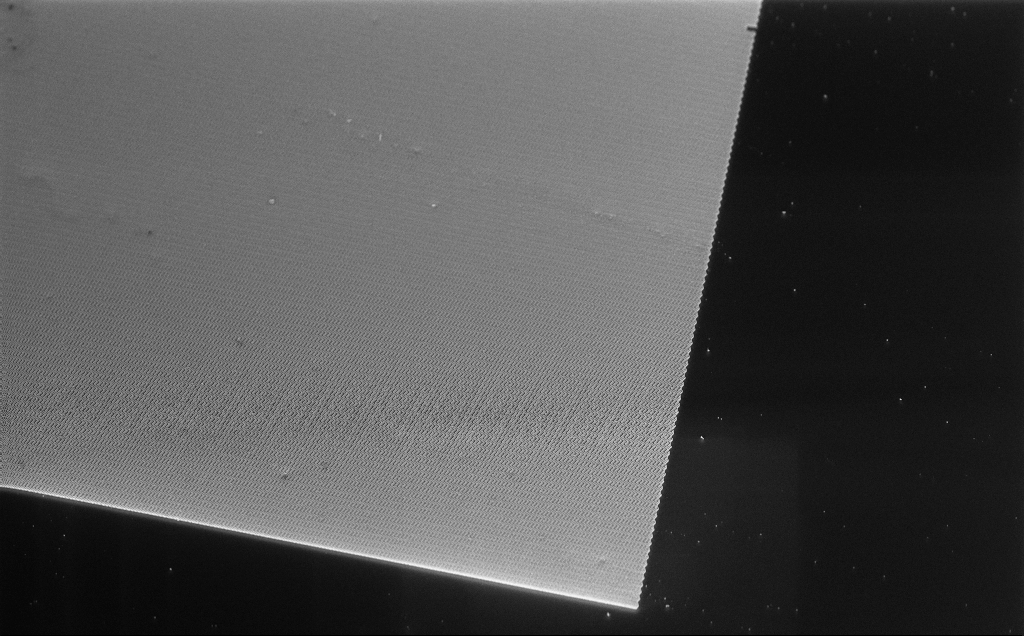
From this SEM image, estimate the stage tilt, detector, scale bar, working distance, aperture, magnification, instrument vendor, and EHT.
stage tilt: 45°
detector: InLens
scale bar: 10000 nm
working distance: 6 mm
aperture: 30 µm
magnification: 1.3 K X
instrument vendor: Zeiss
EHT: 10 kV